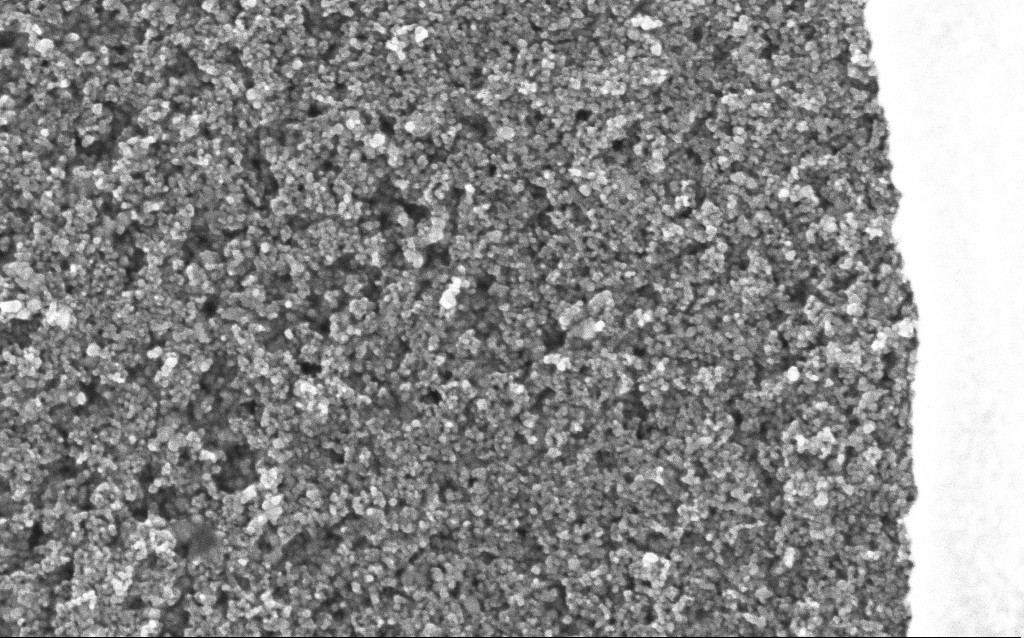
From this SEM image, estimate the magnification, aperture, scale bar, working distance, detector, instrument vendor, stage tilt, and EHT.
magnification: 75.49 K X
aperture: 30 µm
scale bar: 200 nm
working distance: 6.4 mm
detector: InLens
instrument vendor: Zeiss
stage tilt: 0°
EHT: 5 kV